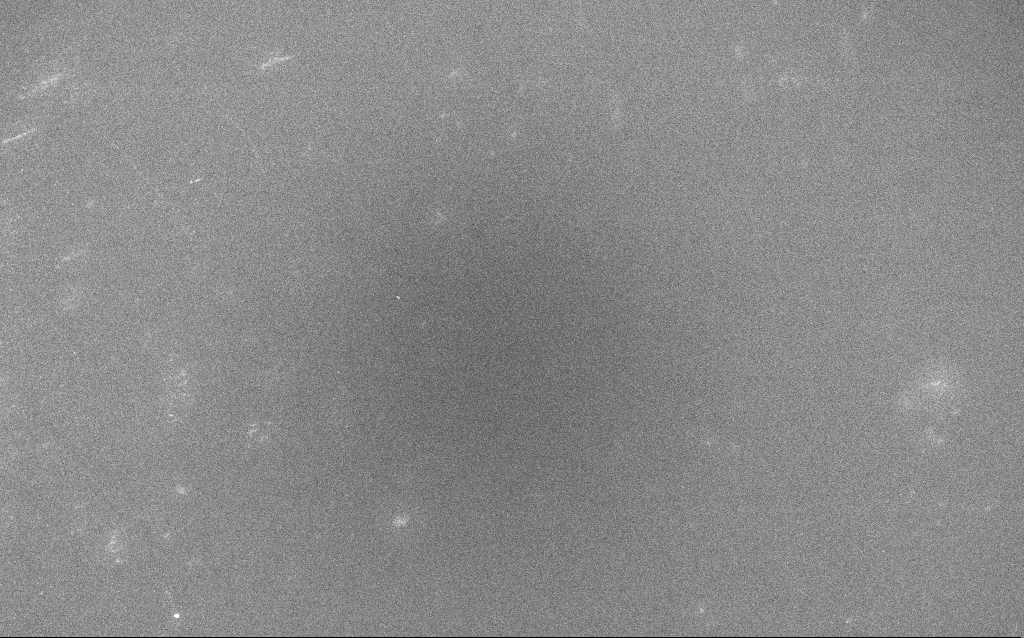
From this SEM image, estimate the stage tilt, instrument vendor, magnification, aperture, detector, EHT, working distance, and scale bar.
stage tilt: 0°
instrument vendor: Zeiss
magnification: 0.25 K X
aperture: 30 µm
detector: InLens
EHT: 20 kV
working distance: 1.4 mm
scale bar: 100000 nm